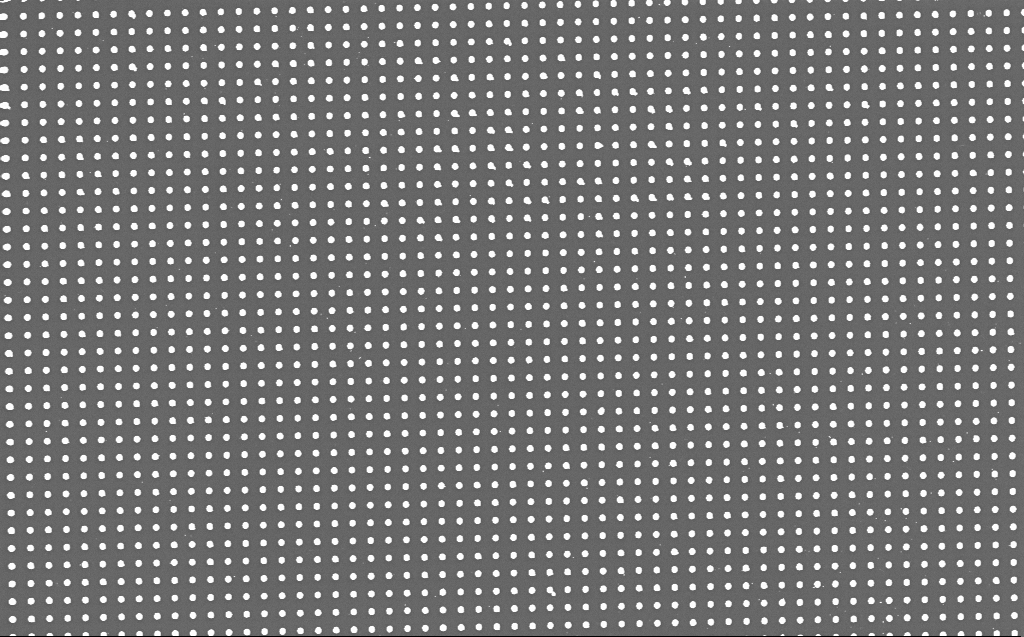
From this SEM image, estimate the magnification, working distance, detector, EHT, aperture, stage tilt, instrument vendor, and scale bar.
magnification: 6.28 K X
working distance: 5 mm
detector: InLens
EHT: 5 kV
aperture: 30 µm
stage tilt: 0°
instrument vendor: Zeiss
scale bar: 10000 nm